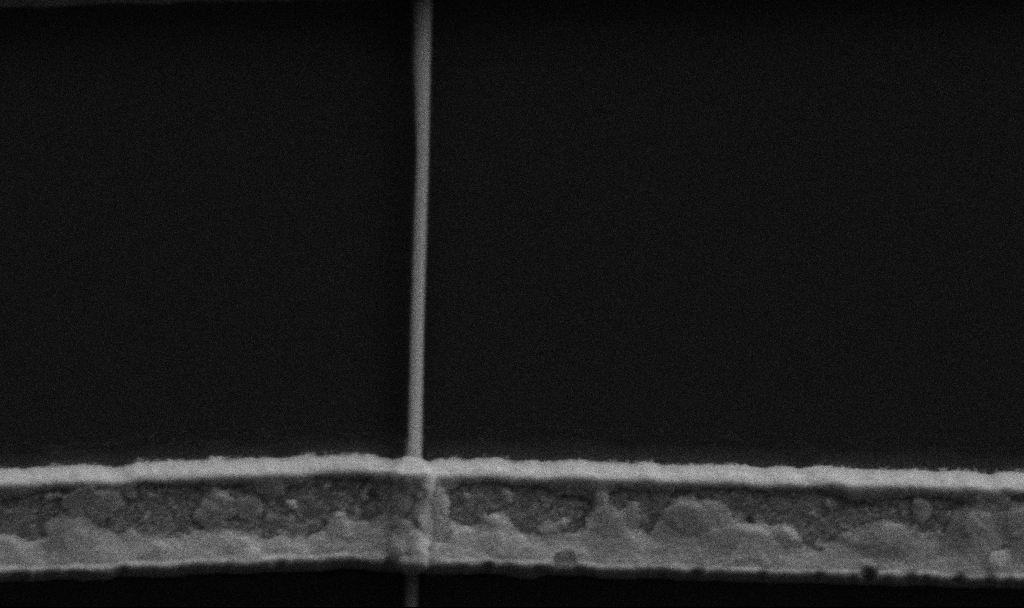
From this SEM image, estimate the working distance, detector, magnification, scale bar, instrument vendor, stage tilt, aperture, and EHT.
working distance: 8.5 mm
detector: SE2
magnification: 60 K X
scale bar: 1000 nm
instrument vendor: Zeiss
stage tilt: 0°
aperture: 30 µm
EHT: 5 kV